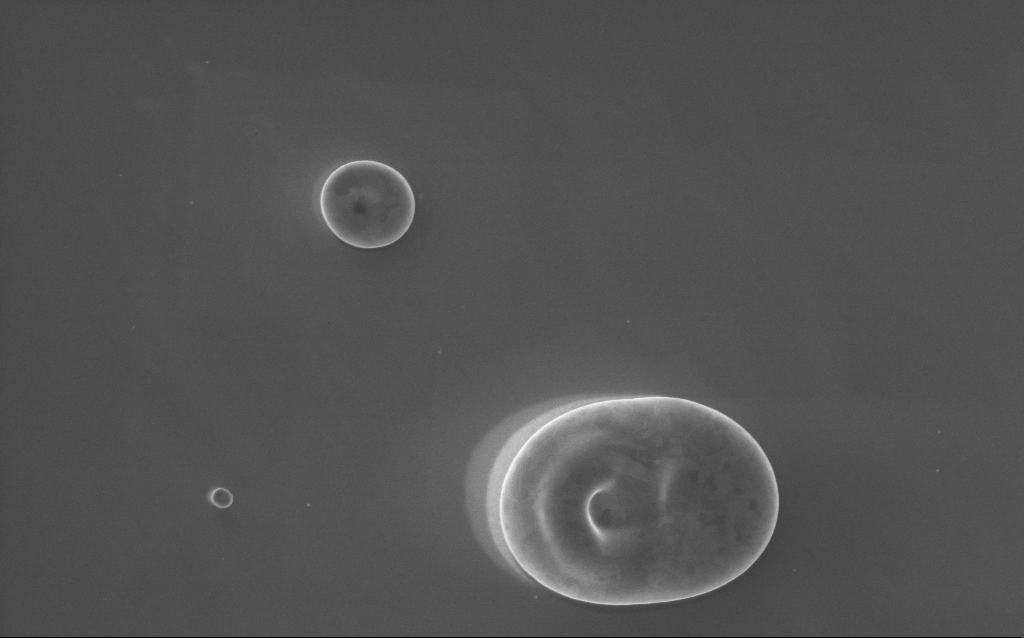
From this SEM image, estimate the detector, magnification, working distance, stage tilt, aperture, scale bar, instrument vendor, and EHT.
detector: InLens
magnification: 20.04 K X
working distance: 3 mm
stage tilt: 0°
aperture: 30 µm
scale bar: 2000 nm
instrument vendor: Zeiss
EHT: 5 kV